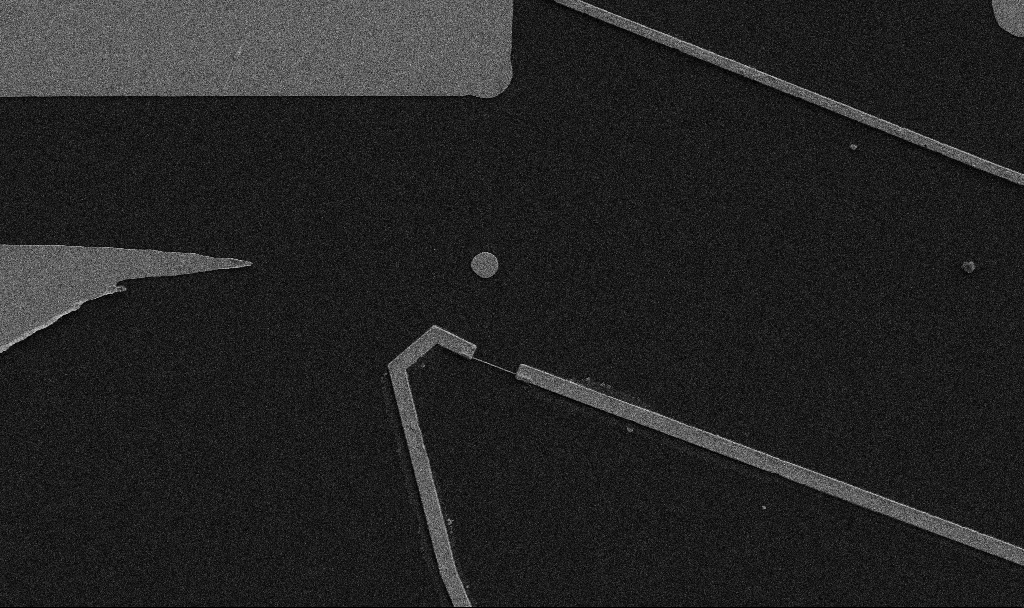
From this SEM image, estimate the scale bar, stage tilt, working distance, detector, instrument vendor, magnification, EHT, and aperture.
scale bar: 10000 nm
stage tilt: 0°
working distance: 10.7 mm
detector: SE2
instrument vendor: Zeiss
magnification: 5 K X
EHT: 5 kV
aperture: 30 µm